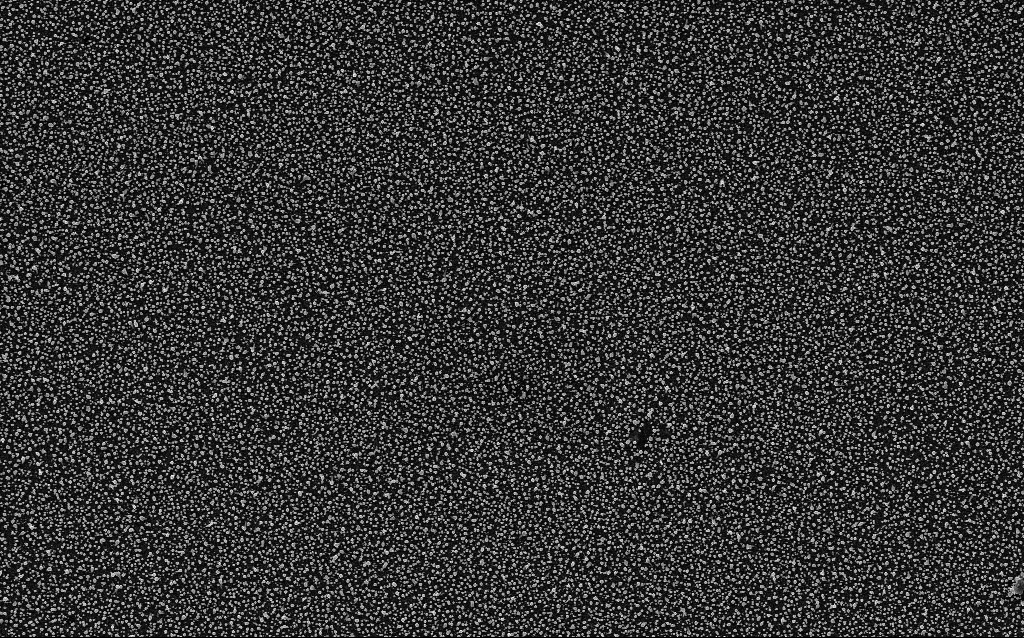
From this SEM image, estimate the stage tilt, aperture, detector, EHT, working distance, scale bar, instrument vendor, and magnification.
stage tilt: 0°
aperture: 30 µm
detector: InLens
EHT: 5 kV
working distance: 2.1 mm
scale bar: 2000 nm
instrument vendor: Zeiss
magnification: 10 K X